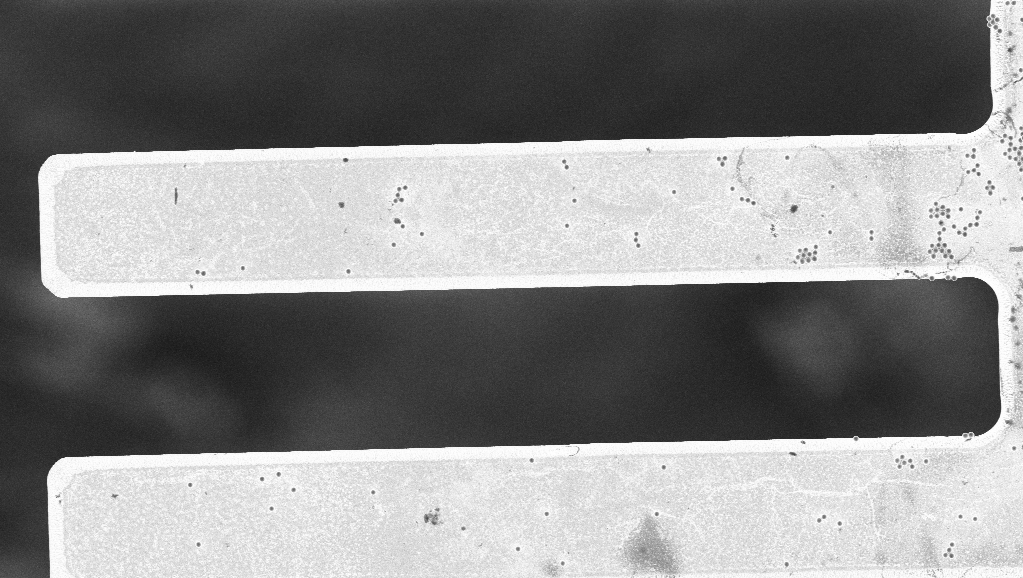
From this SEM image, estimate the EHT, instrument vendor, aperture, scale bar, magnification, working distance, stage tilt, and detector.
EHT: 3 kV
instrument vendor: Zeiss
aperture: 30 µm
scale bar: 20000 nm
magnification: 2.8 K X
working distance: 7 mm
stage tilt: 0°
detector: InLens